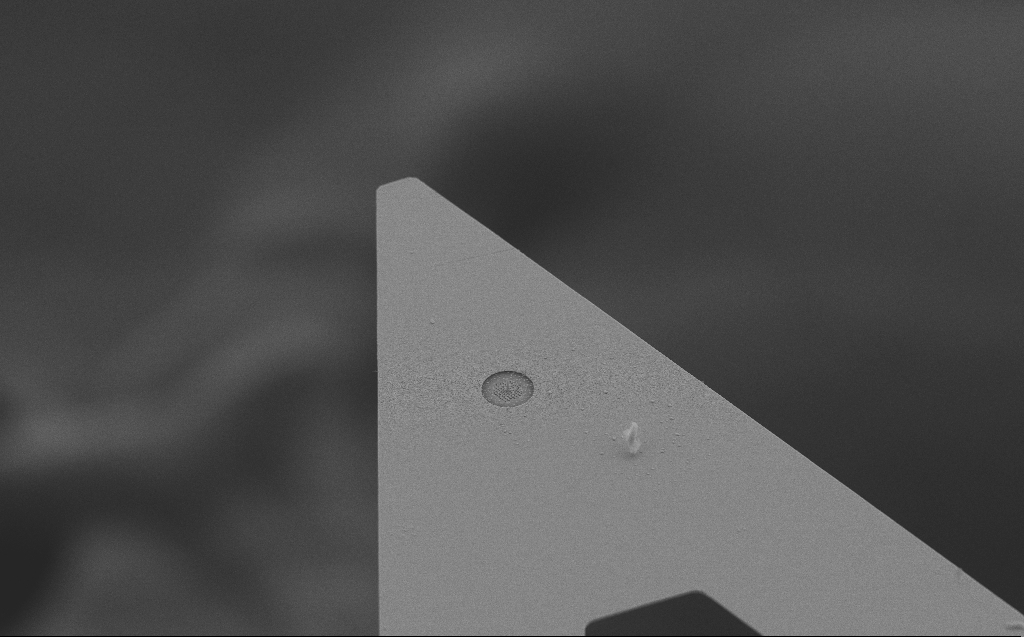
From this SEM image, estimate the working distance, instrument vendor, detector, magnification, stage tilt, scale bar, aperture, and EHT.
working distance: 6 mm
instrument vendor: Zeiss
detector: SE2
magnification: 2.76 K X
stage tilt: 45°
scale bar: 20000 nm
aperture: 30 µm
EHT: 10 kV